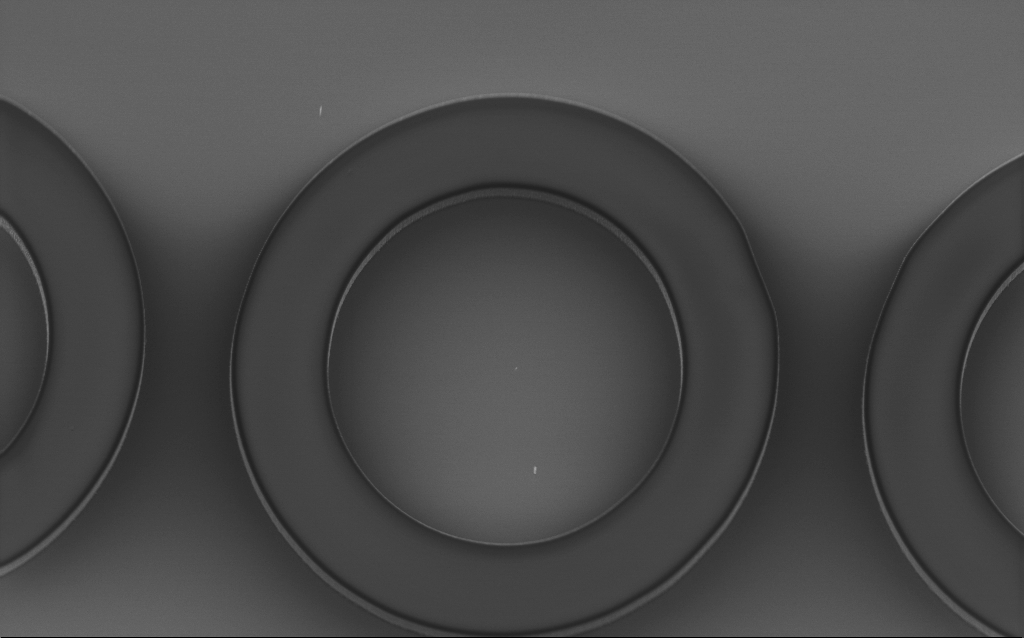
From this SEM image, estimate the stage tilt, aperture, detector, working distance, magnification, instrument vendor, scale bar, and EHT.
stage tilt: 45°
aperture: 30 µm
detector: InLens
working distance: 3.4 mm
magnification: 3.37 K X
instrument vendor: Zeiss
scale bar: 20000 nm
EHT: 2 kV